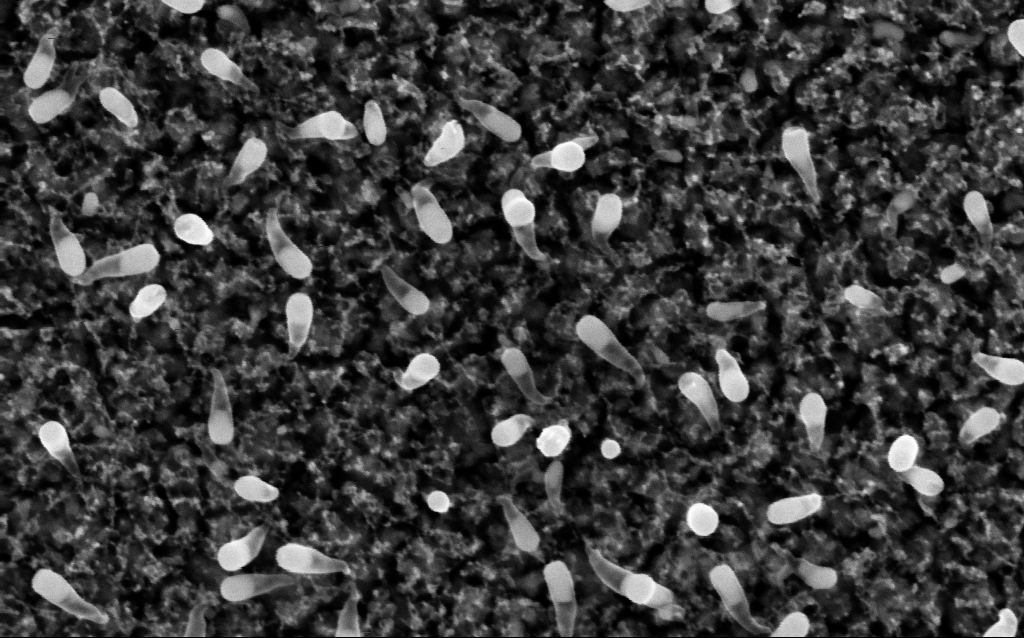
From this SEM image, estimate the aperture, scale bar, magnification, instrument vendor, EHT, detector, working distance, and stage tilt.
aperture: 30 µm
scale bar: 100 nm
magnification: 200 K X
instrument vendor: Zeiss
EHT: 5 kV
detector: InLens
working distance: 1.8 mm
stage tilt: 0°